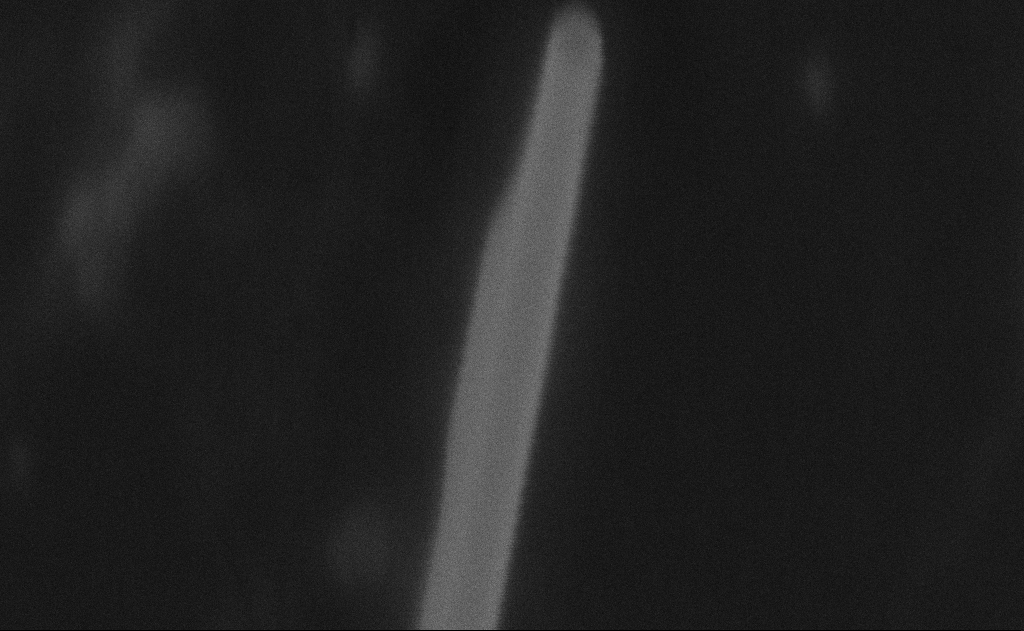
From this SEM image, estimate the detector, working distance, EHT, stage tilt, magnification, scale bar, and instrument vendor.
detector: SE2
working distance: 11 mm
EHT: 20 kV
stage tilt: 0°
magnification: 447.46 K X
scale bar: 100 nm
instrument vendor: Zeiss